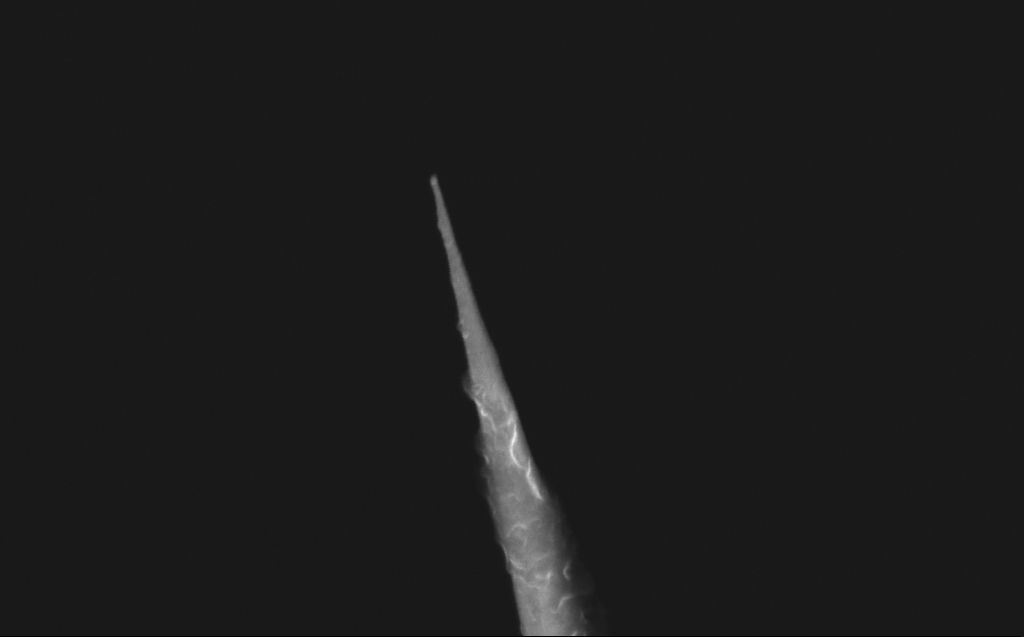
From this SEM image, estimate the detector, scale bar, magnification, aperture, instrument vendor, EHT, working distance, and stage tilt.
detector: InLens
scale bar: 200 nm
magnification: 88.08 K X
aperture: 30 µm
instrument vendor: Zeiss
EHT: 10 kV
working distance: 6 mm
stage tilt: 40°